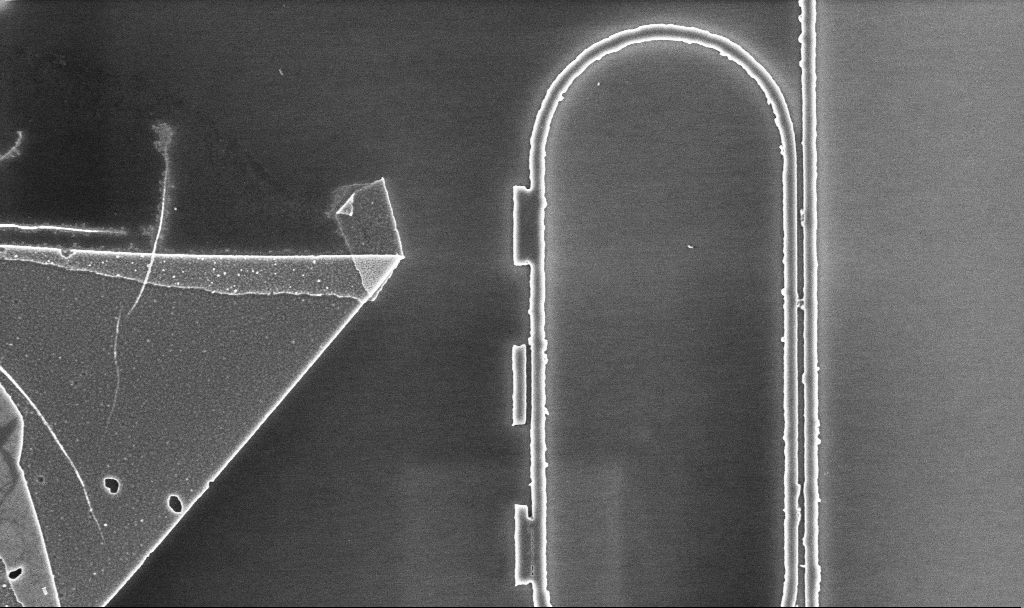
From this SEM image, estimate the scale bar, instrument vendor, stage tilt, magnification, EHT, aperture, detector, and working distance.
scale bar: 2000 nm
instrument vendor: Zeiss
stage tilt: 0°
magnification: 9.91 K X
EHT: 5 kV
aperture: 30 µm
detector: InLens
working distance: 5.2 mm